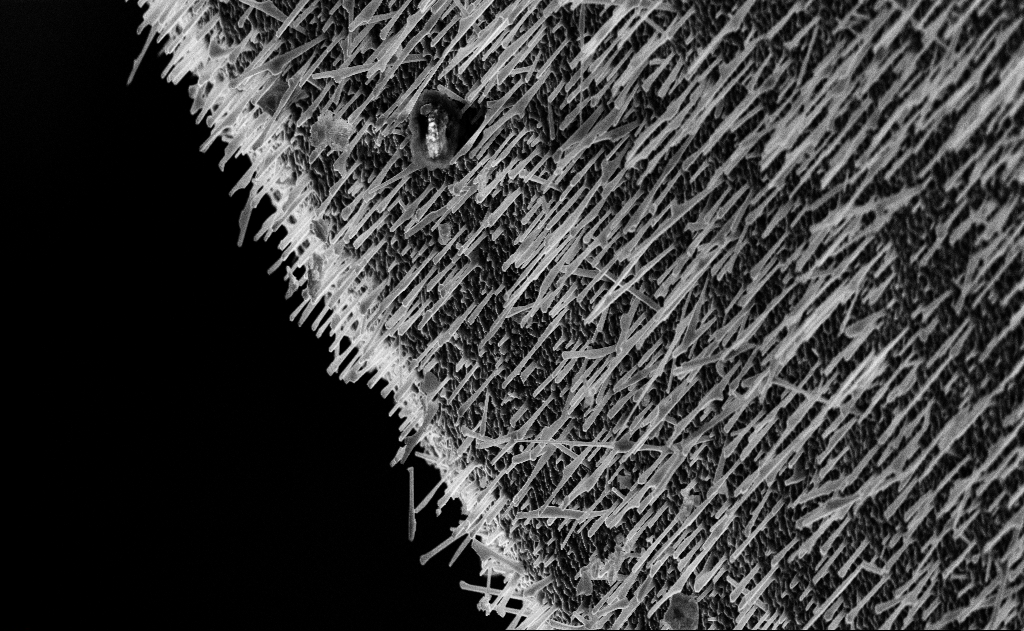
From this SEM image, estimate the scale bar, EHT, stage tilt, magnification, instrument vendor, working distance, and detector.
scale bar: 10000 nm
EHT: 10 kV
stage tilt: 0°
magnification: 5 K X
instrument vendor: Zeiss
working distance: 15 mm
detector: InLens